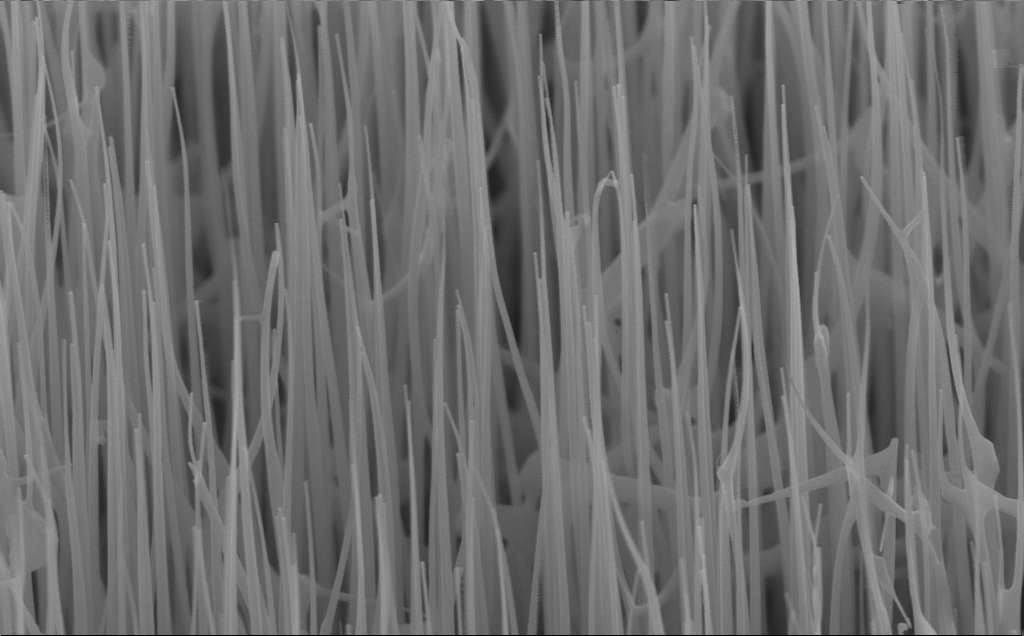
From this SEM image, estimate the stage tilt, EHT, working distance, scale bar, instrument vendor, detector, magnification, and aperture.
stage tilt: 45°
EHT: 10 kV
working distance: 6 mm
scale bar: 200 nm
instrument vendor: Zeiss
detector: InLens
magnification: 80 K X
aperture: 30 µm